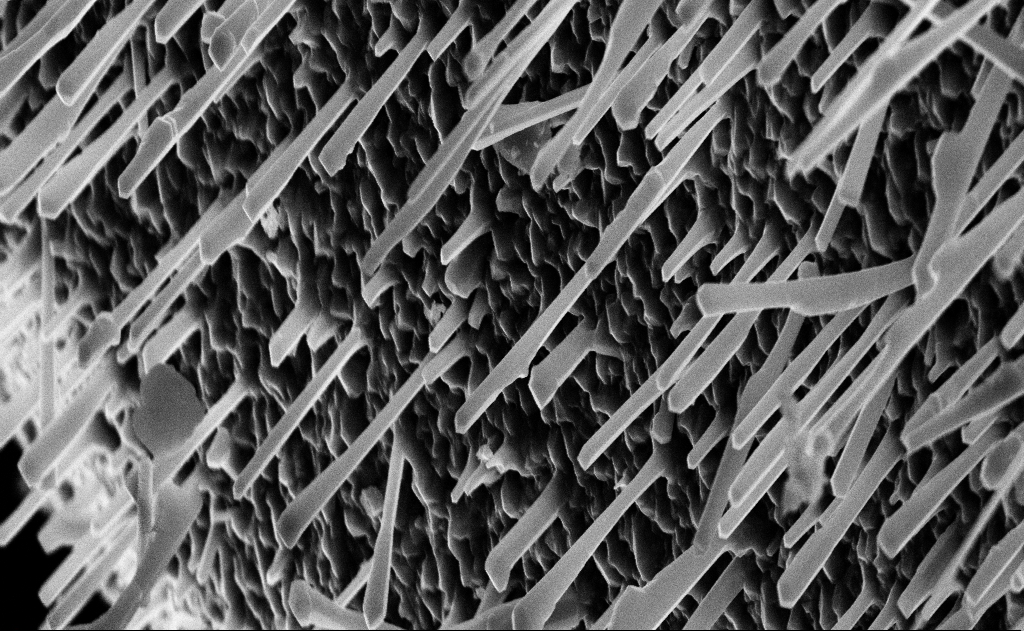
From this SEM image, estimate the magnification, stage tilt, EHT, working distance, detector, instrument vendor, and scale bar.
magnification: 20 K X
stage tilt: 0°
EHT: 10 kV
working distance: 15 mm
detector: InLens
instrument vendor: Zeiss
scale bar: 2000 nm